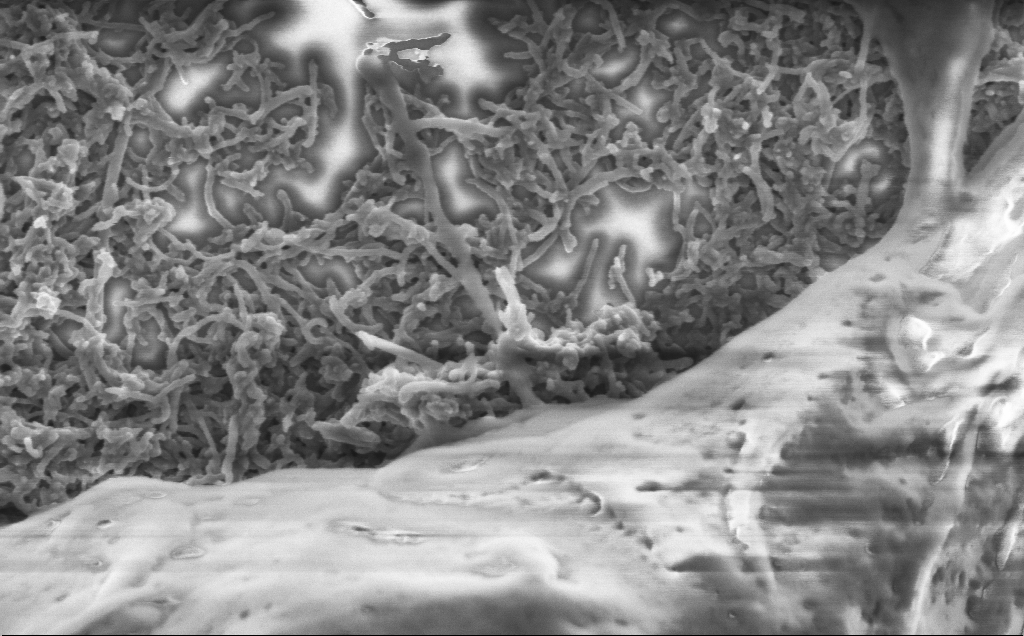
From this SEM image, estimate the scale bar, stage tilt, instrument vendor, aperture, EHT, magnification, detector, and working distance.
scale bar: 200 nm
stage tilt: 0°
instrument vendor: Zeiss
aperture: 30 µm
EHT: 2 kV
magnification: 75 K X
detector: InLens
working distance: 7.1 mm